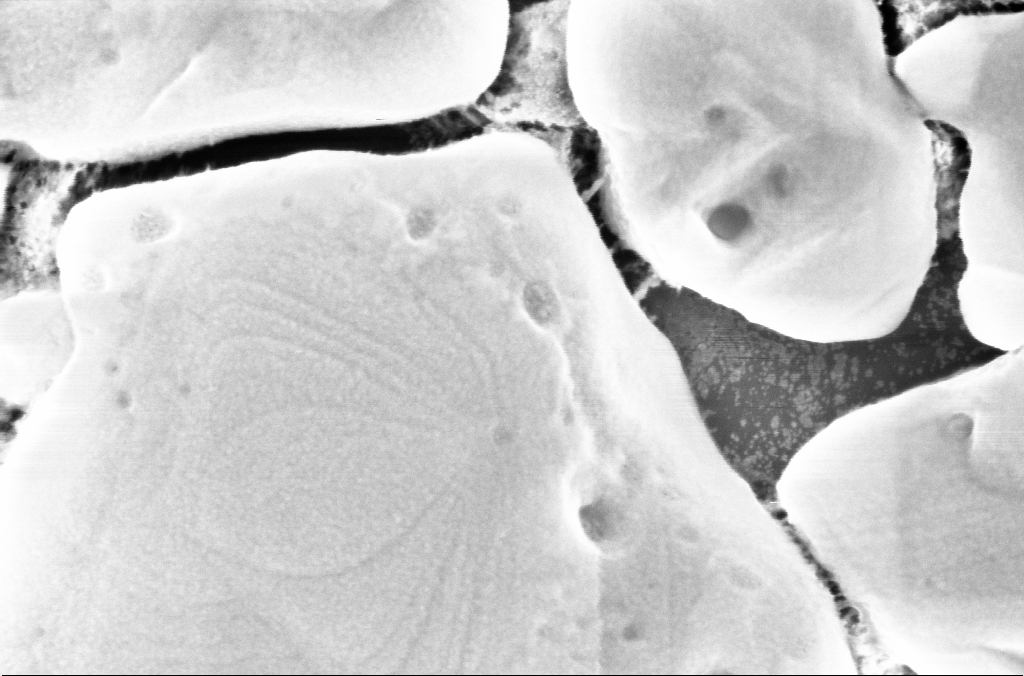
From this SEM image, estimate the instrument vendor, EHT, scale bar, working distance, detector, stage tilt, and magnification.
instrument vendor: Zeiss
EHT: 5 kV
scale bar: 200 nm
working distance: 3 mm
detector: InLens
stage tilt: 0°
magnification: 189.21 K X